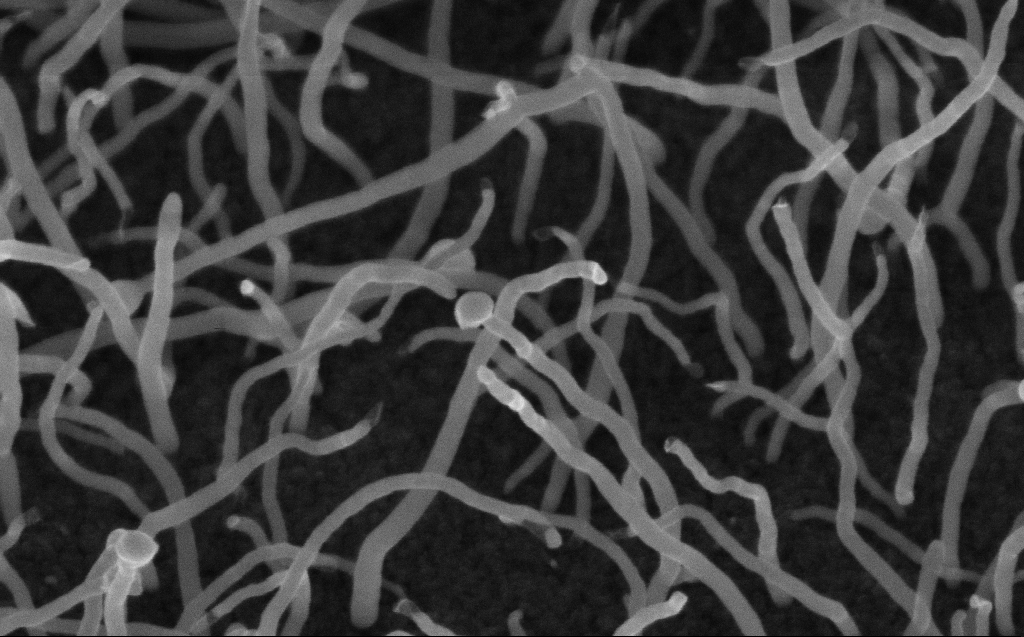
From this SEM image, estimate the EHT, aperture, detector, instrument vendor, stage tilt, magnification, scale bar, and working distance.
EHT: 10 kV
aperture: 30 µm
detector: InLens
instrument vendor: Zeiss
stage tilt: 45°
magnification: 144.5 K X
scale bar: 200 nm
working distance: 6 mm